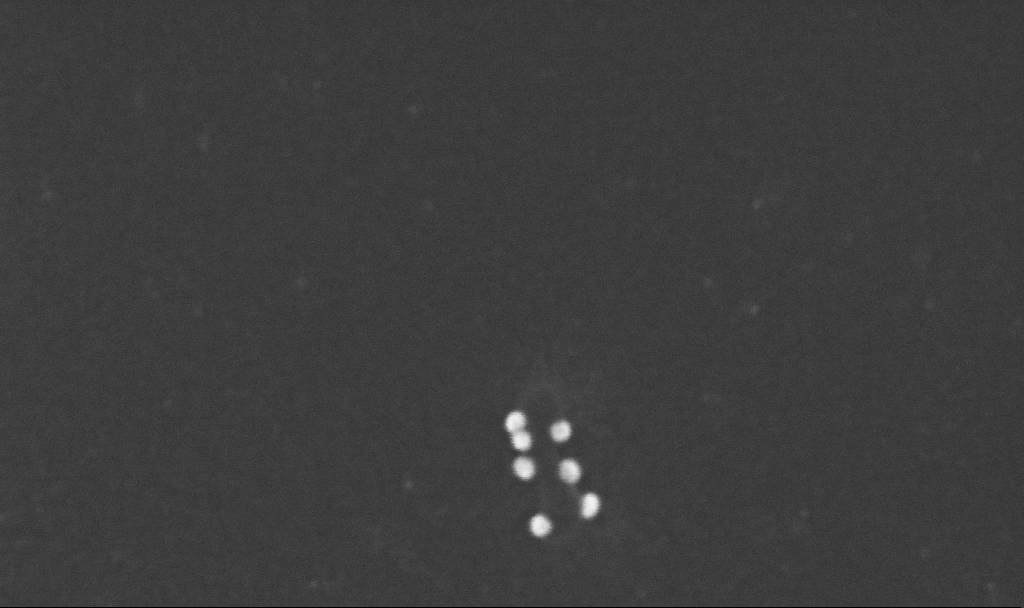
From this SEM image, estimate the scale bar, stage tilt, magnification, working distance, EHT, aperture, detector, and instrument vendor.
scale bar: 200 nm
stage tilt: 0°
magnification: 356.98 K X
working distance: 3.2 mm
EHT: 10 kV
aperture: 30 µm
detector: InLens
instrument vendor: Zeiss